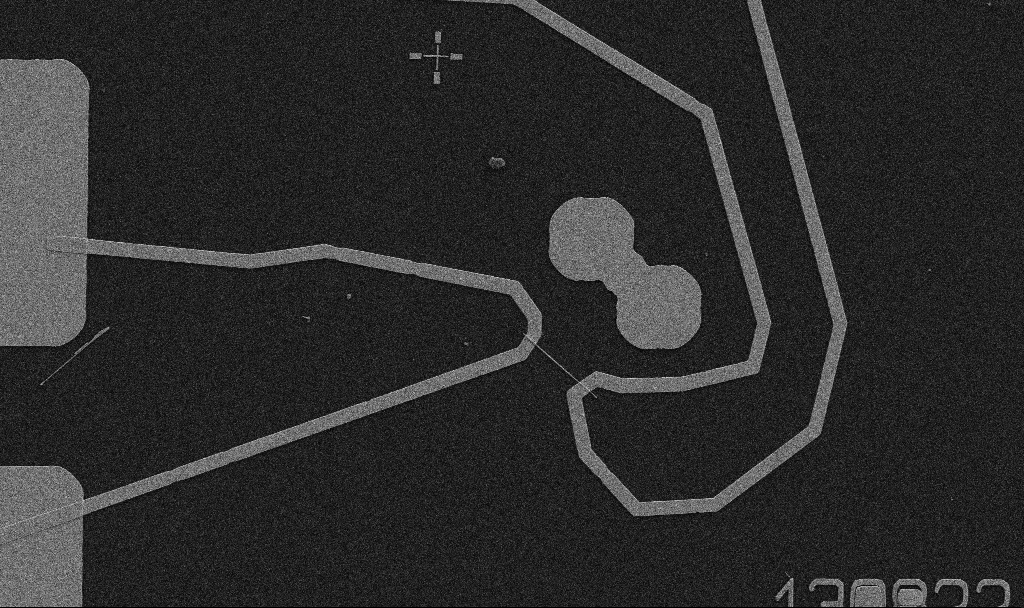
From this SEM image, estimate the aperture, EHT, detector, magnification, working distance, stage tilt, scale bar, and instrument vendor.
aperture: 30 µm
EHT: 5 kV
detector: SE2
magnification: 5 K X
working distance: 10.7 mm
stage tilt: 0°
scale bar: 10000 nm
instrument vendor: Zeiss